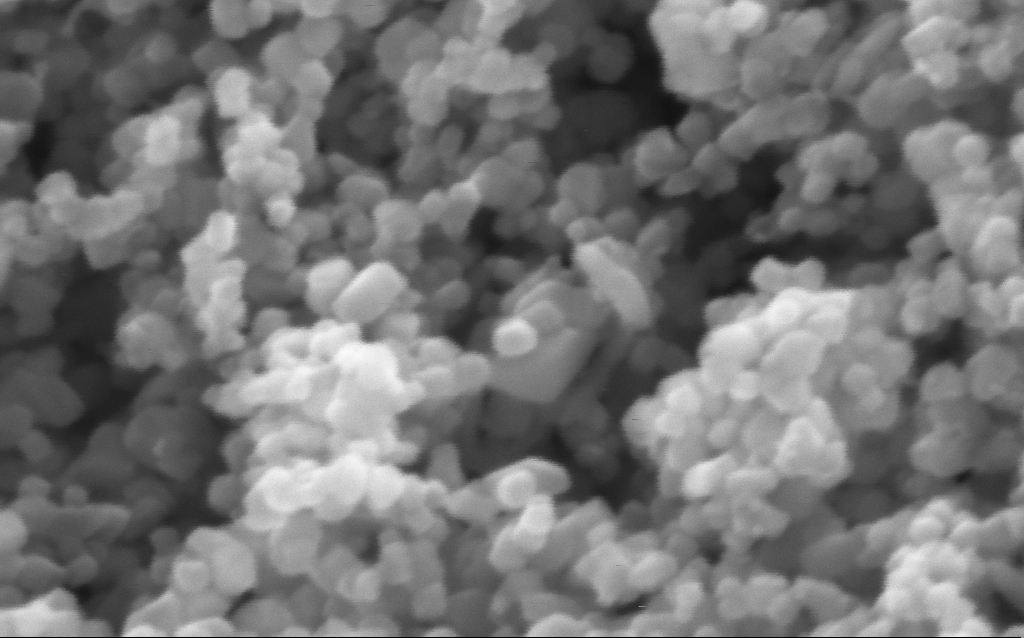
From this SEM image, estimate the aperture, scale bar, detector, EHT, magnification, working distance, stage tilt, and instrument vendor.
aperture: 30 µm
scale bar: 100 nm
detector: InLens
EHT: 5 kV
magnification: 600 K X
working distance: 4.4 mm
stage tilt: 0°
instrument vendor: Zeiss